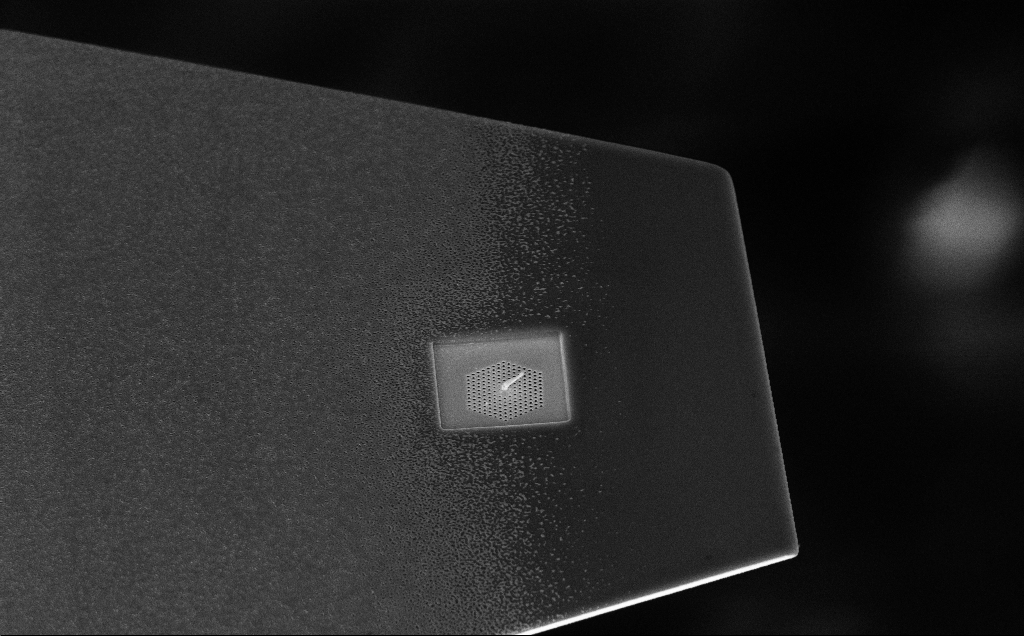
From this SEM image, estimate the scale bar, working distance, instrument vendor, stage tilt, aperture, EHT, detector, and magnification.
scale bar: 2000 nm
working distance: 11 mm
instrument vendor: Zeiss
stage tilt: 45°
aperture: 30 µm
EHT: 10 kV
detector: InLens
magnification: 7.56 K X